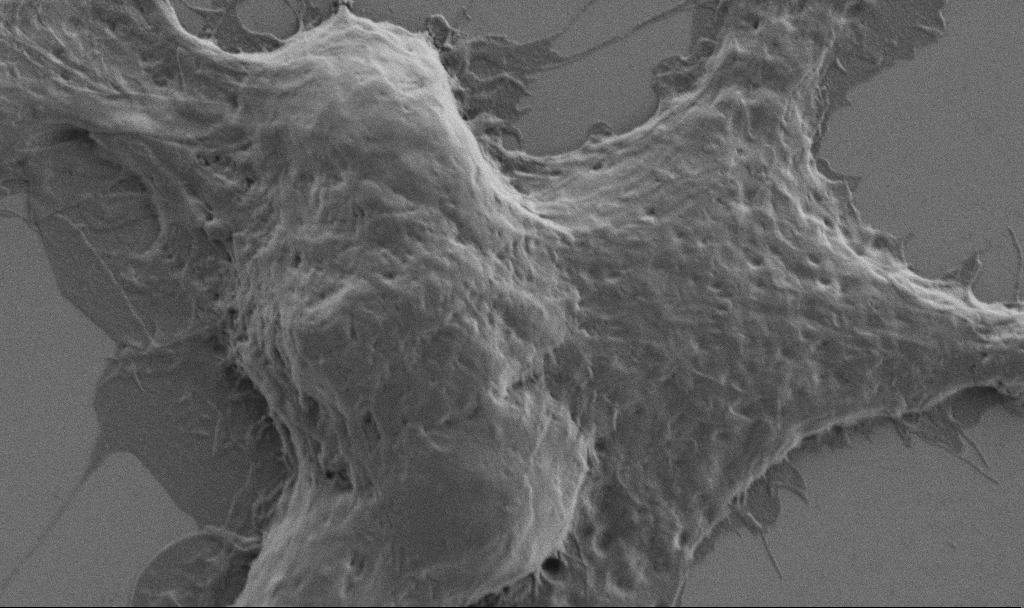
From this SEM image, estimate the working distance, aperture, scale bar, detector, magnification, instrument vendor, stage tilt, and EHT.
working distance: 6.9 mm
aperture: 30 µm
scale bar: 2000 nm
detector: SE2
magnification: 10 K X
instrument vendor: Zeiss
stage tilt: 0°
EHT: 1 kV